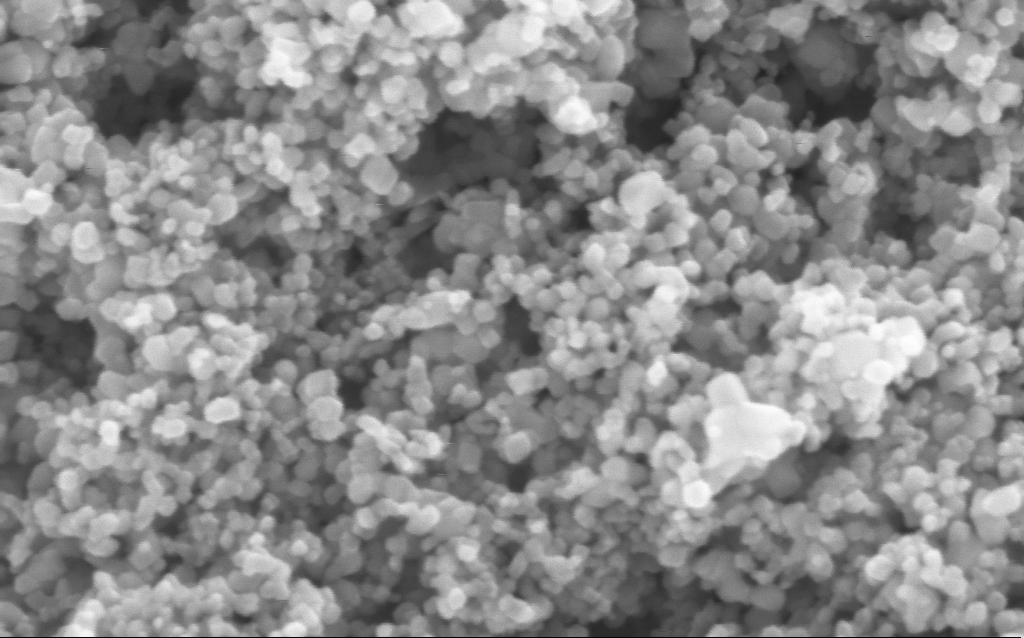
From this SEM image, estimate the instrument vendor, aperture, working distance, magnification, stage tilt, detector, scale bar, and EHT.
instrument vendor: Zeiss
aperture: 30 µm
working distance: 4.4 mm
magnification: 294.51 K X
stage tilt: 0°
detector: InLens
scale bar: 200 nm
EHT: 5 kV